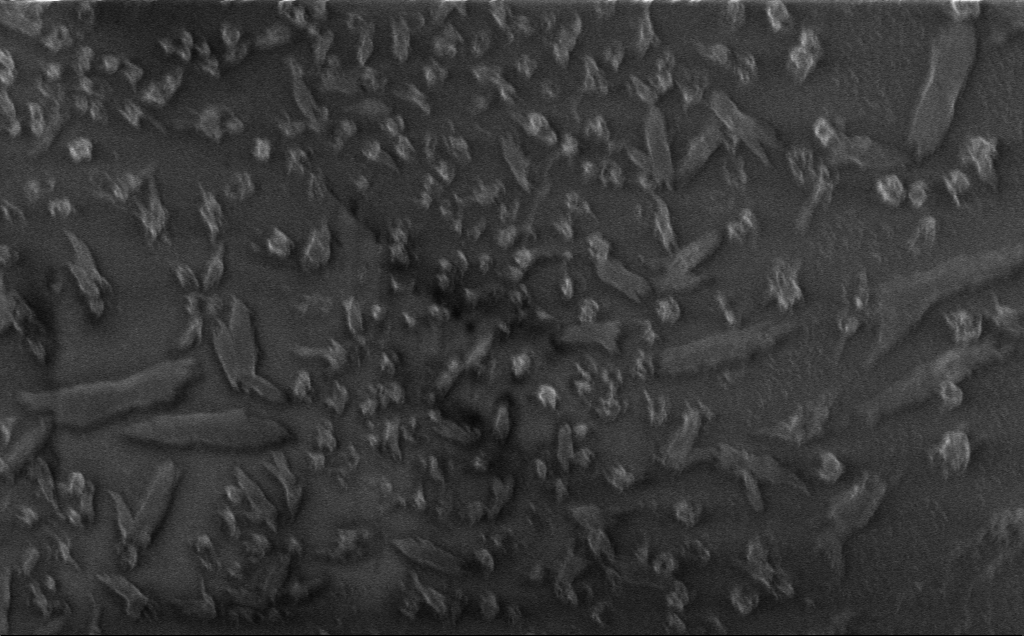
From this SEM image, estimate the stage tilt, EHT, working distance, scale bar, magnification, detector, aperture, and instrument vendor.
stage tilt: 0°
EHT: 1 kV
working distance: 3 mm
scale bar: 2000 nm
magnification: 9.29 K X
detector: InLens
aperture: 30 µm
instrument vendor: Zeiss